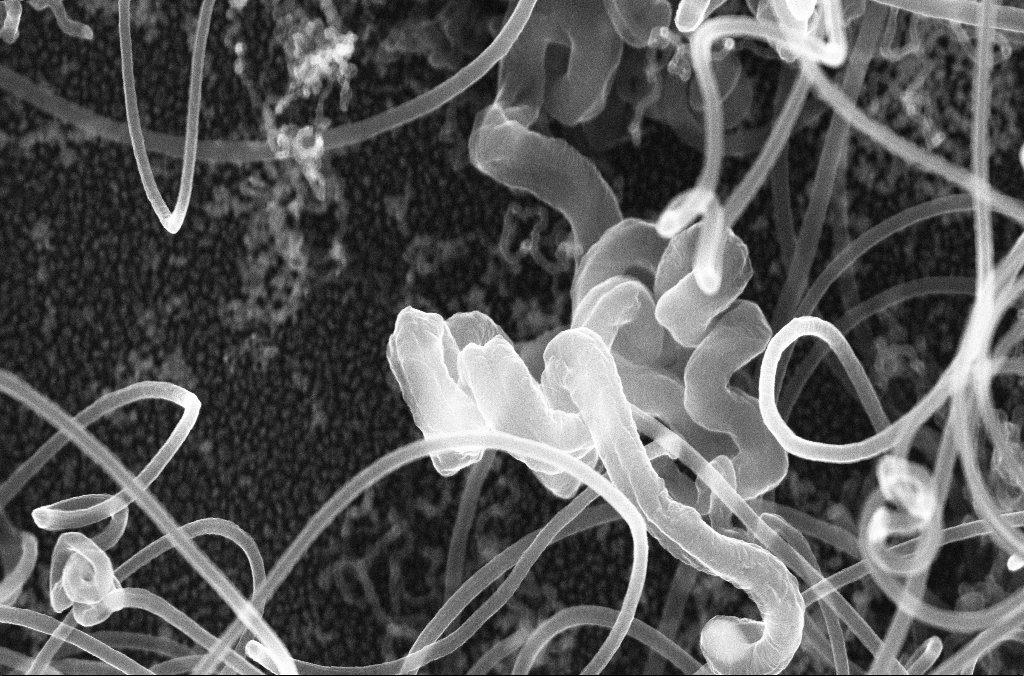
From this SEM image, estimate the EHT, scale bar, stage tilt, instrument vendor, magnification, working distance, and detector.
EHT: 20 kV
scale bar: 1000 nm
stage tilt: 0°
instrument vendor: Zeiss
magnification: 50 K X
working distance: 4.2 mm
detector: InLens